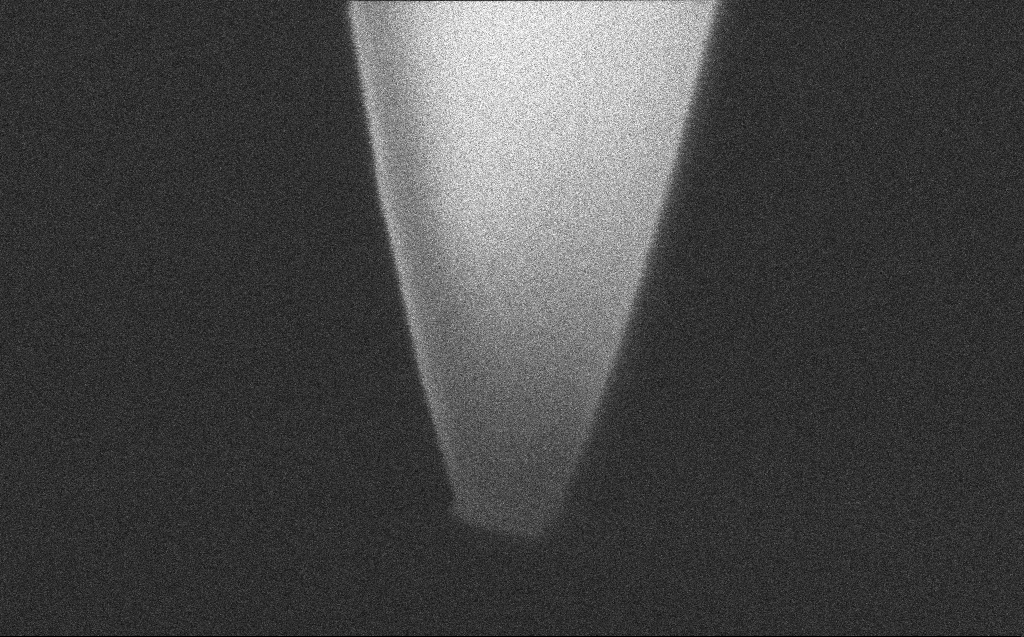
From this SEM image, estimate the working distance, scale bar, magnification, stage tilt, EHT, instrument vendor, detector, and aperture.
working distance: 3 mm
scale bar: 100 nm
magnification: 420.51 K X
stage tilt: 45.1°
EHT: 2 kV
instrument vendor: Zeiss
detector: InLens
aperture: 30 µm